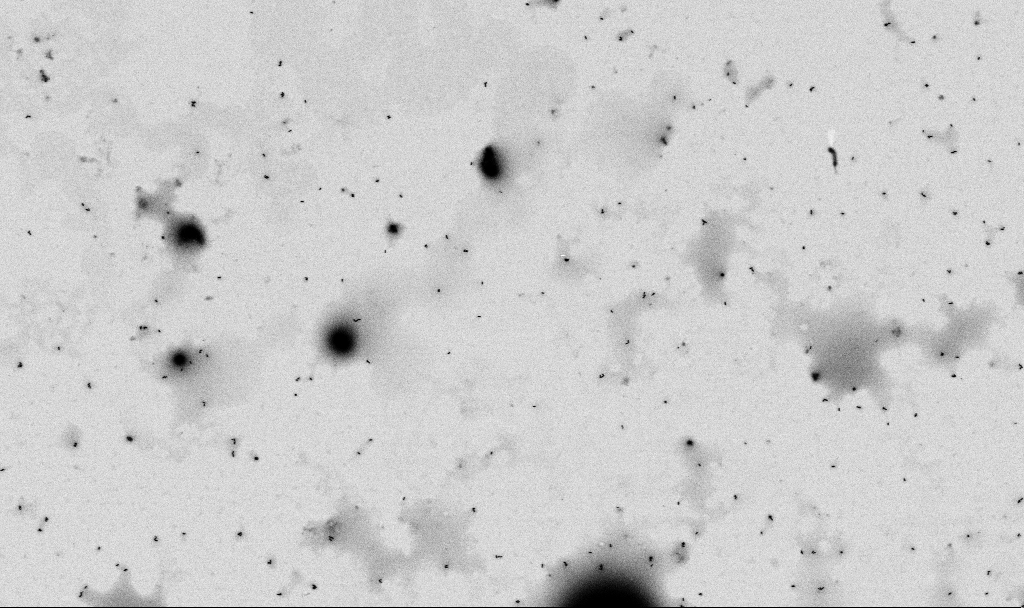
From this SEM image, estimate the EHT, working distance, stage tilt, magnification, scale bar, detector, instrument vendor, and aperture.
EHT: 2 kV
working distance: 4.5 mm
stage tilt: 0°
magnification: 13.03 K X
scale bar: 1000 nm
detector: SE2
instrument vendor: Zeiss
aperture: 30 µm